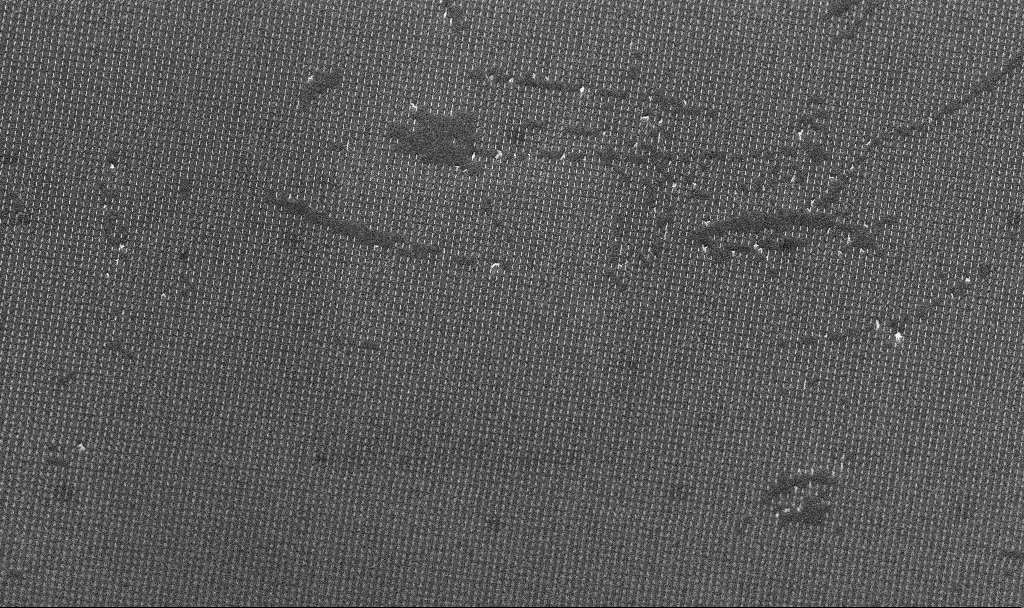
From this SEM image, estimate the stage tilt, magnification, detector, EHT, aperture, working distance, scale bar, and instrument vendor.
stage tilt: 45°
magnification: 11.19 K X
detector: InLens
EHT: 5 kV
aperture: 30 µm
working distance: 7.4 mm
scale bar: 2000 nm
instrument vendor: Zeiss